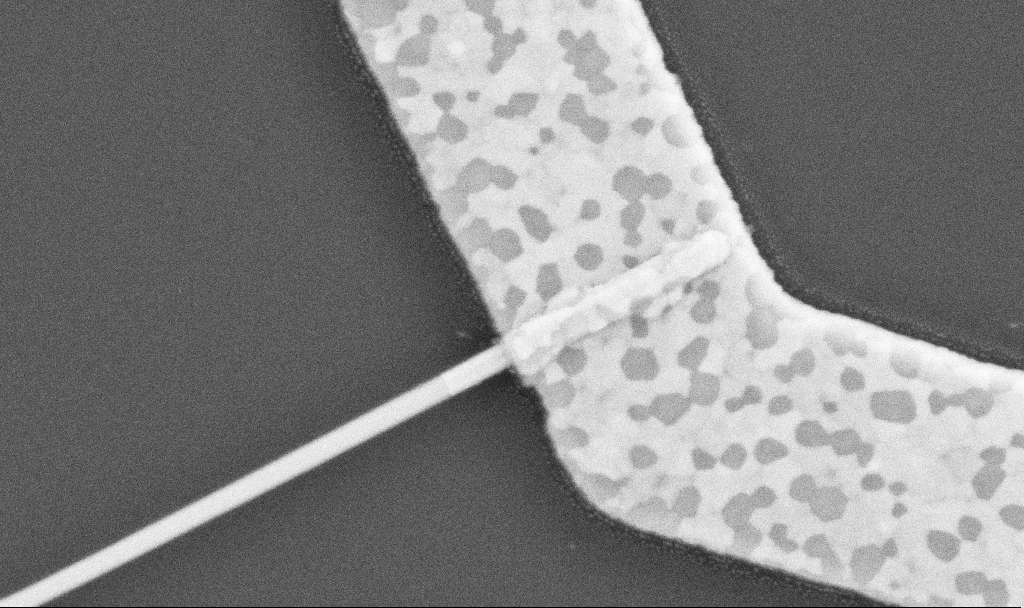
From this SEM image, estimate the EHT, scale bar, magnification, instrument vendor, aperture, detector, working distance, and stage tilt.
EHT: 5 kV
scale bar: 200 nm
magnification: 100 K X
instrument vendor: Zeiss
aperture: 30 µm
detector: SE2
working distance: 10.5 mm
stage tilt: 0°